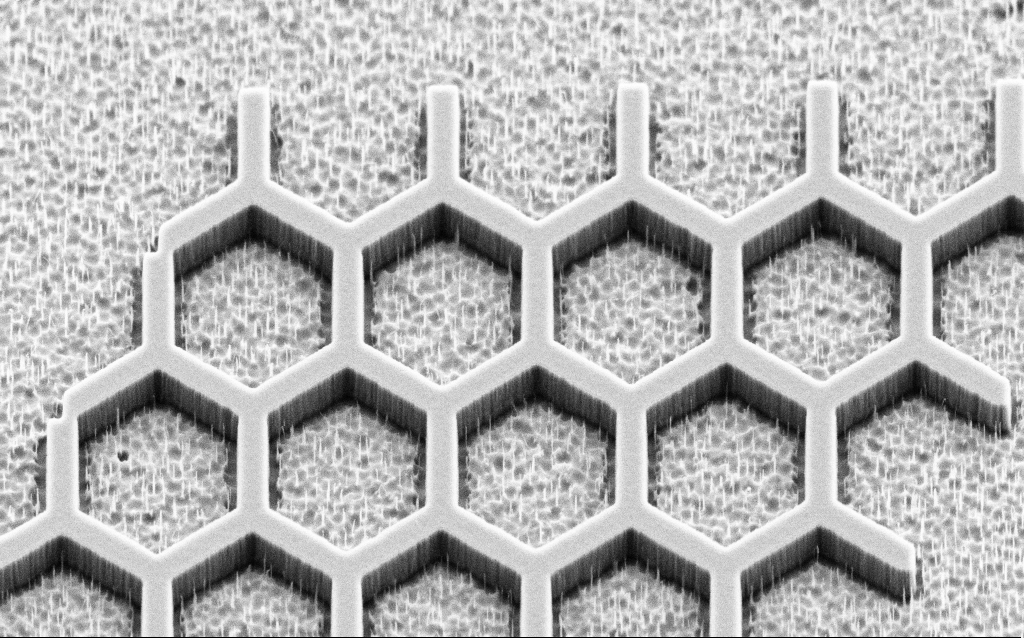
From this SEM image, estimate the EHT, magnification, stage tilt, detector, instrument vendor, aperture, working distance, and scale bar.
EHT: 3 kV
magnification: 22.41 K X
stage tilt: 45°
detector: SE2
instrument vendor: Zeiss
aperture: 30 µm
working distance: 8 mm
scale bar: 2000 nm